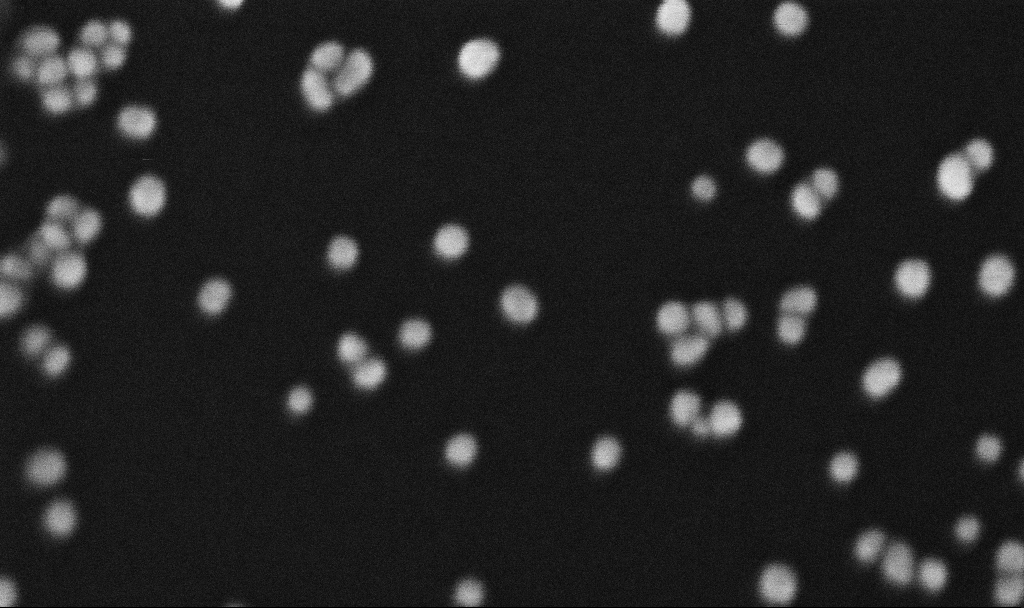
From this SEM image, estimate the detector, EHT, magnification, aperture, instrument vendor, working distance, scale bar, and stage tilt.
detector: InLens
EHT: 10 kV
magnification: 573.58 K X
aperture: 30 µm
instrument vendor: Zeiss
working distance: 5.4 mm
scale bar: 100 nm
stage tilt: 0°